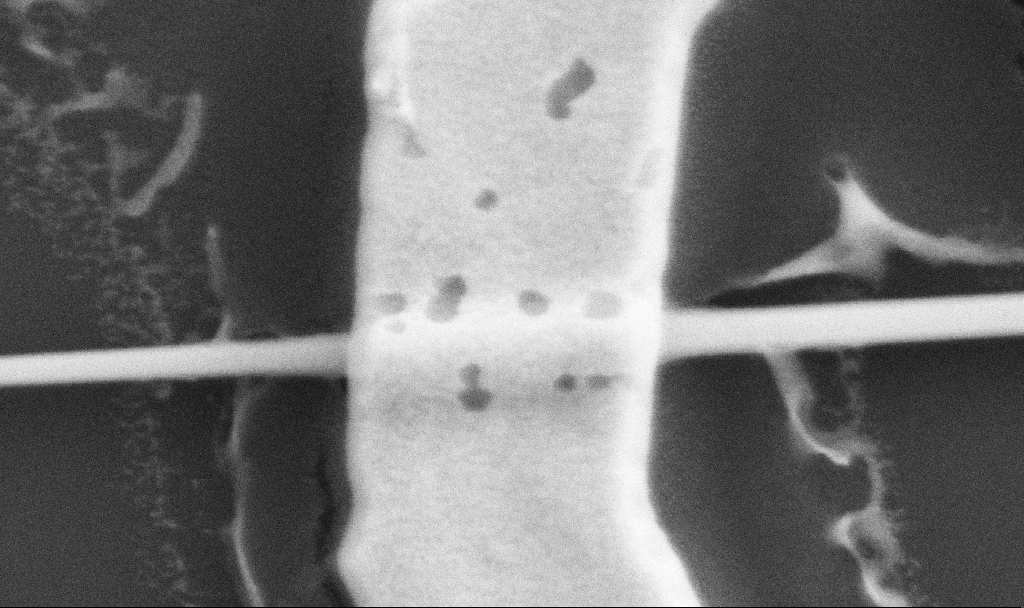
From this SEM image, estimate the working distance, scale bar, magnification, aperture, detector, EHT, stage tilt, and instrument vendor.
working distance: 10.7 mm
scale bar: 100 nm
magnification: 150 K X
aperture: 30 µm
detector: SE2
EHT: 5 kV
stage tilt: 0°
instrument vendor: Zeiss